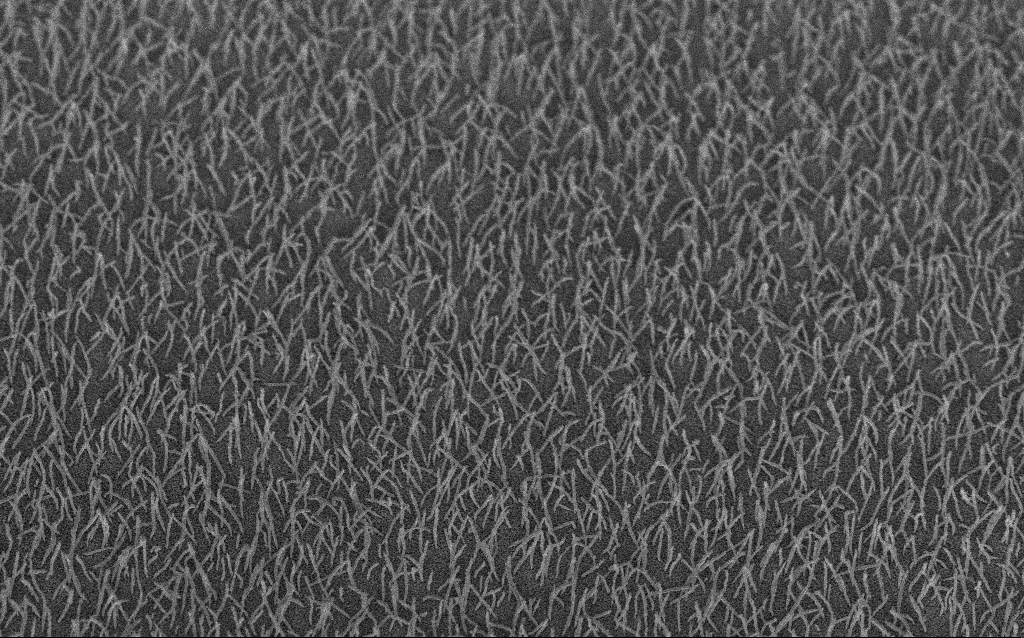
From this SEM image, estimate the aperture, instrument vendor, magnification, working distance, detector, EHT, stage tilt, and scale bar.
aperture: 30 µm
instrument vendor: Zeiss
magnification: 10 K X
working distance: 8.2 mm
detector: InLens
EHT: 5 kV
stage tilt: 45°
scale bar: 2000 nm